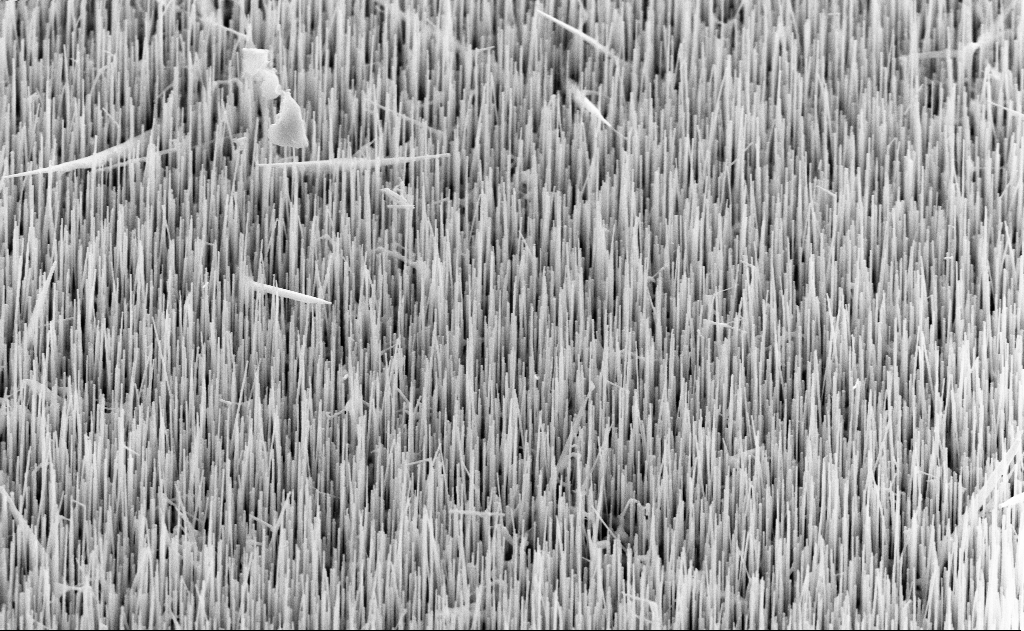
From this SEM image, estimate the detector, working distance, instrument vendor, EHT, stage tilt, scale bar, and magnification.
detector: SE2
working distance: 11 mm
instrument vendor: Zeiss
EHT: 10 kV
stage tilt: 45°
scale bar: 1000 nm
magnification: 20 K X